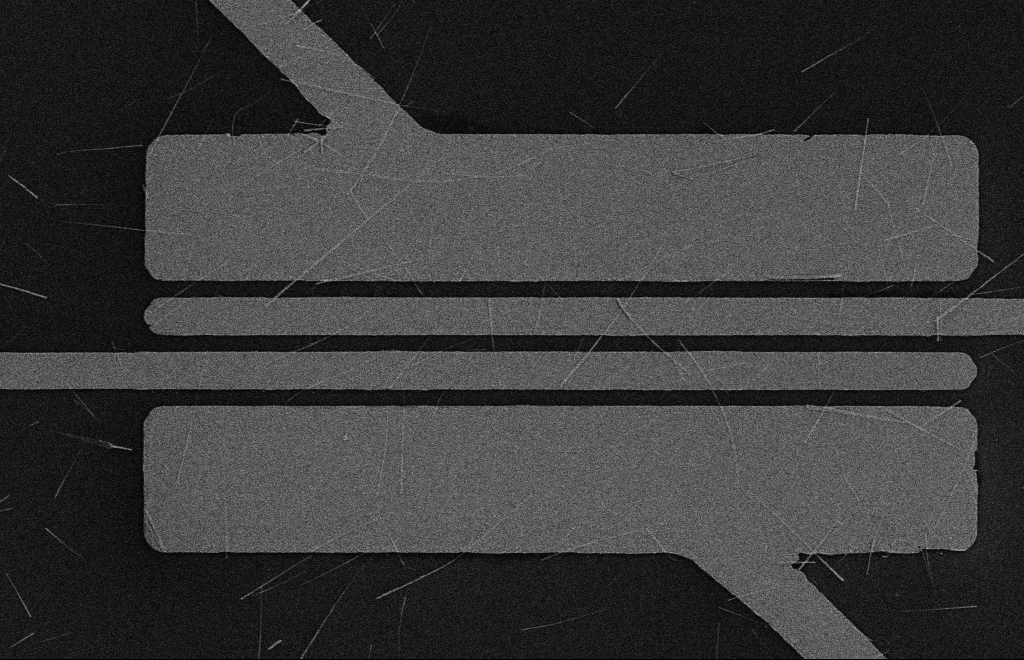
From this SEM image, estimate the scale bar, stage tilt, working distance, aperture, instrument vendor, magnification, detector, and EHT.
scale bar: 2000 nm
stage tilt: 0°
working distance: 16 mm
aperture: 10 µm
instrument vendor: Zeiss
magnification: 5.03 K X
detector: SE2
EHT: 5 kV